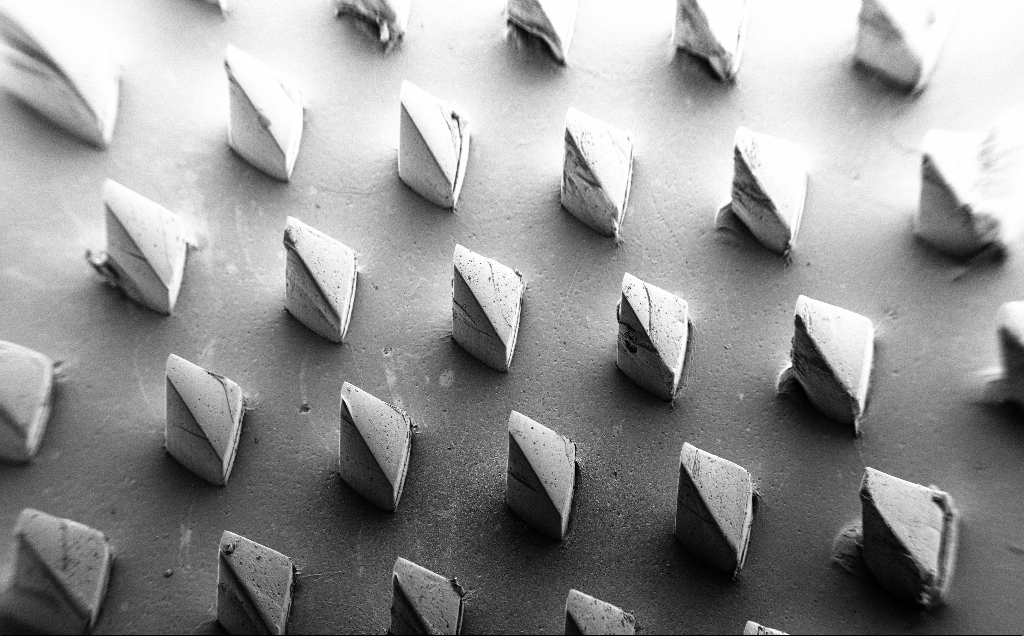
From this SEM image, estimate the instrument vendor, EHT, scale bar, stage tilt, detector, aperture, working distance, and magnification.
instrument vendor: Zeiss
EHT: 5 kV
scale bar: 1e+06 nm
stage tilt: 39°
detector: SE2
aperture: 30 µm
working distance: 8 mm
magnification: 0.067 K X